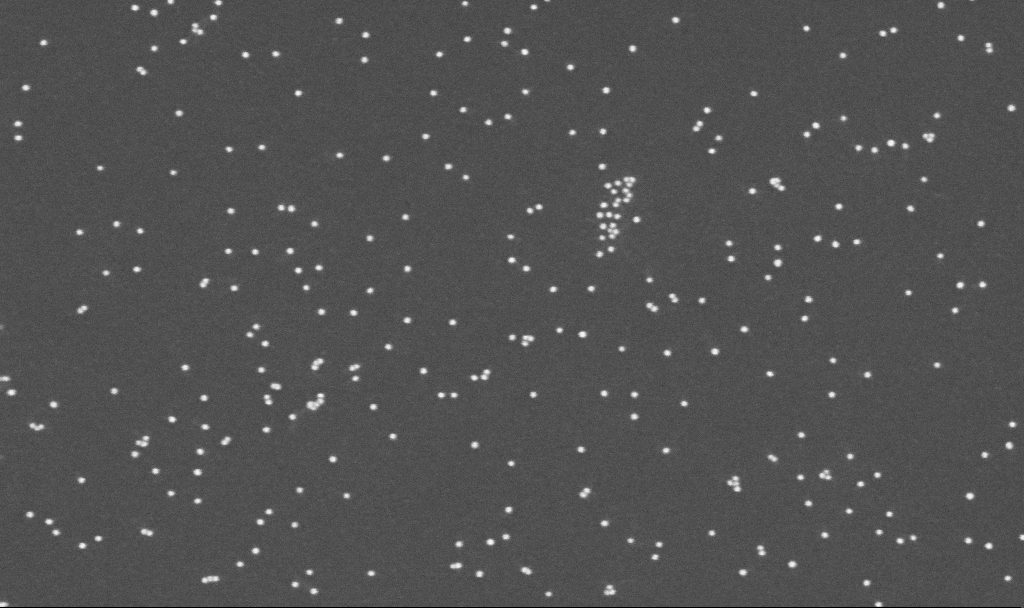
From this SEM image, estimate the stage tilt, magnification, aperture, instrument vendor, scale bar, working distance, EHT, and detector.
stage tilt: -0°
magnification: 200 K X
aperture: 30 µm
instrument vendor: Zeiss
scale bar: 200 nm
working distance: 3.3 mm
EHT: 10 kV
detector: InLens